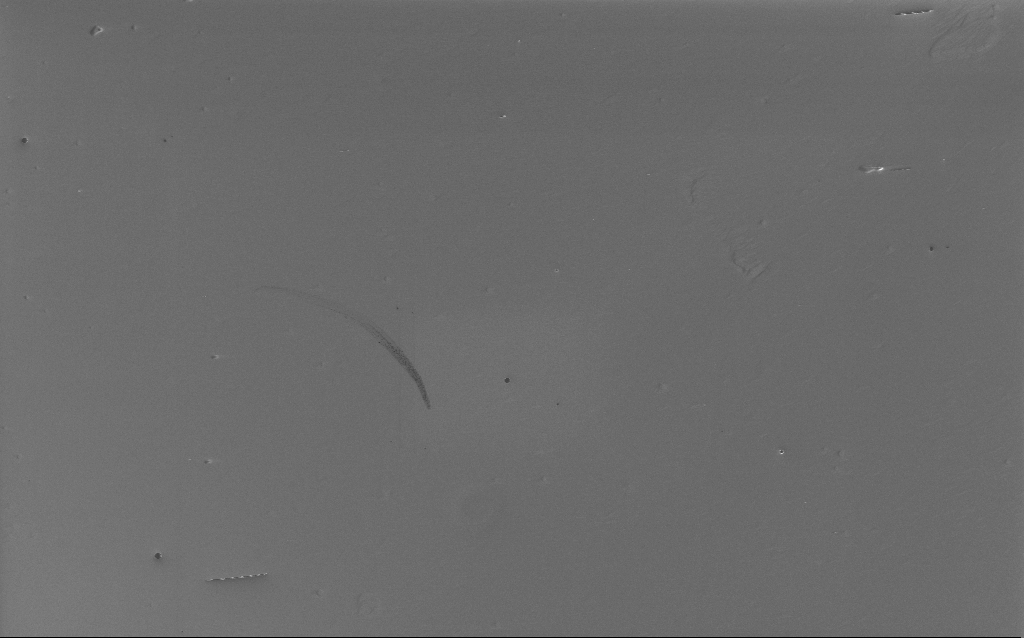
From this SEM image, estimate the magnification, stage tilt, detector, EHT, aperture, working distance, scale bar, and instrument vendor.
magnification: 1.5 K X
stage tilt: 0°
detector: InLens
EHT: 5 kV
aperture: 30 µm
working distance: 4 mm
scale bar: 10000 nm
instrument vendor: Zeiss